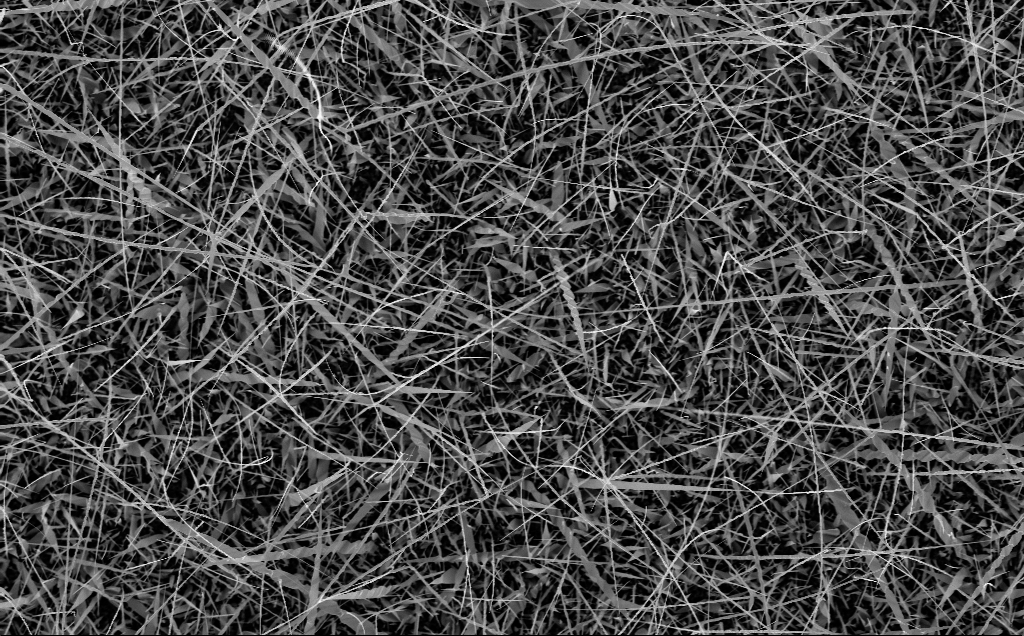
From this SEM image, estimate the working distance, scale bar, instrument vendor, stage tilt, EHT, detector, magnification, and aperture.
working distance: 6 mm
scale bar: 10000 nm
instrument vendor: Zeiss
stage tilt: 0°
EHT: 10 kV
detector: InLens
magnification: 5 K X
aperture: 30 µm